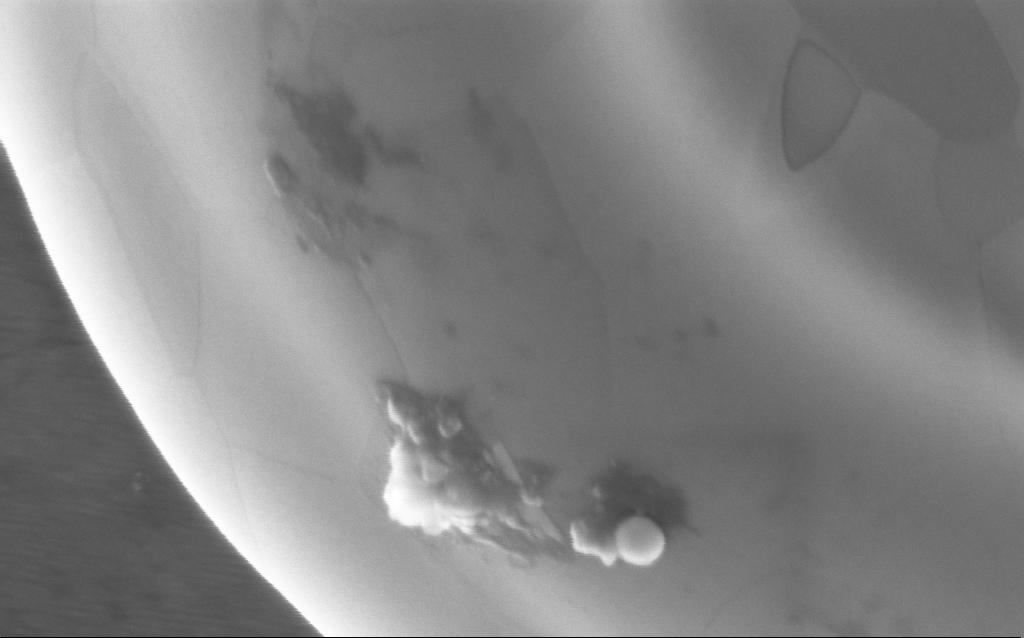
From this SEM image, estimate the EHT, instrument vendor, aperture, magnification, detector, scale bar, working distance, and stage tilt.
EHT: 5 kV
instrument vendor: Zeiss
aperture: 30 µm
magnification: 199.88 K X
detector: InLens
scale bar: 200 nm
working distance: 4 mm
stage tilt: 0°